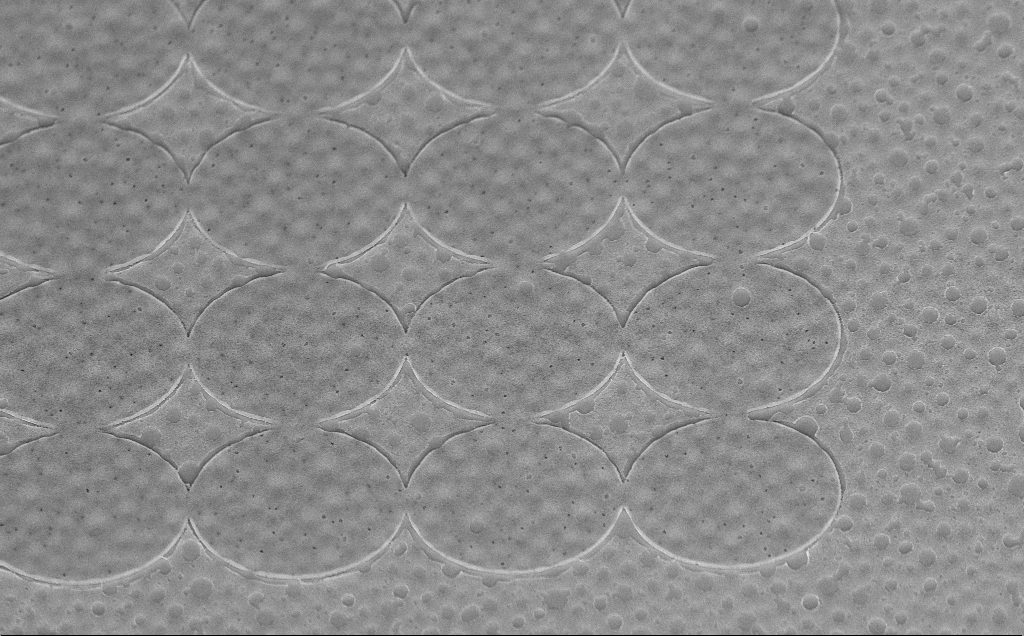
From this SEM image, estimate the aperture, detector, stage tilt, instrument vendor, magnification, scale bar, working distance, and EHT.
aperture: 30 µm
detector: InLens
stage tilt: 45°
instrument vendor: Zeiss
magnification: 8.05 K X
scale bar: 2000 nm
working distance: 6 mm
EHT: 5 kV